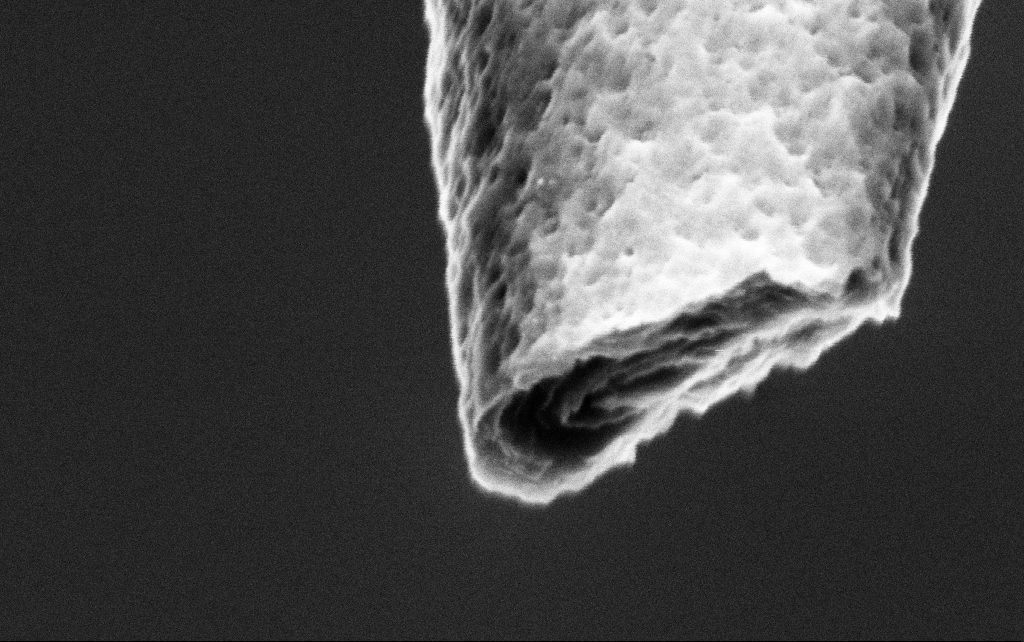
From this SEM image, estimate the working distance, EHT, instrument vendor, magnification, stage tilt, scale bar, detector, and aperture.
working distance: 7.7 mm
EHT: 3 kV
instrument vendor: Zeiss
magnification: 150 K X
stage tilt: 45°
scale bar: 200 nm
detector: InLens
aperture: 30 µm